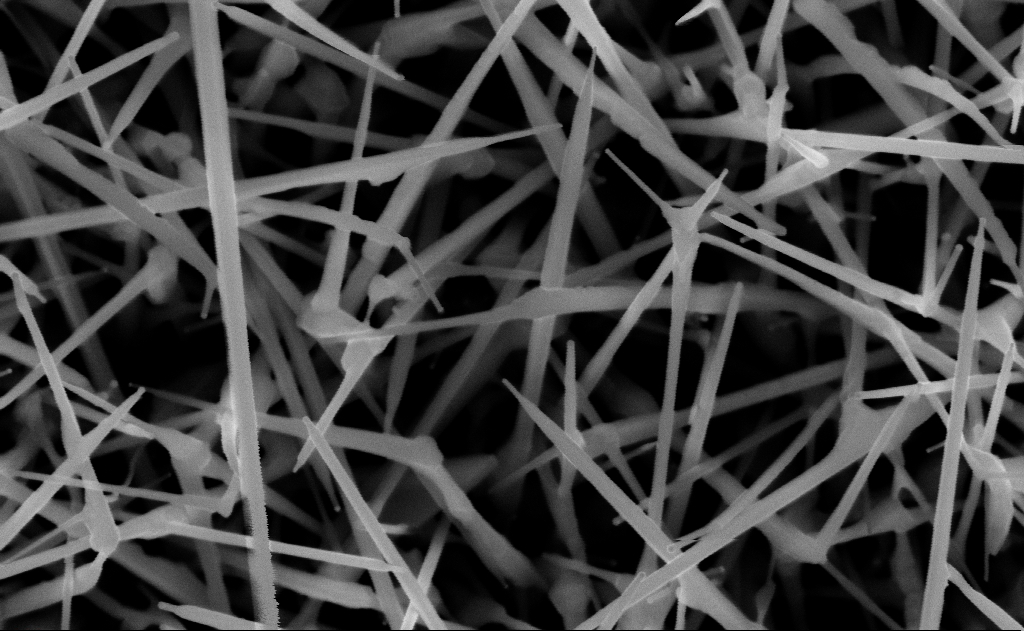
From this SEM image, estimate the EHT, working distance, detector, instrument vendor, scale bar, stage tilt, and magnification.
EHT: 10 kV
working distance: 13 mm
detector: InLens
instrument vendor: Zeiss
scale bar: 200 nm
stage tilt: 0°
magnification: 80 K X